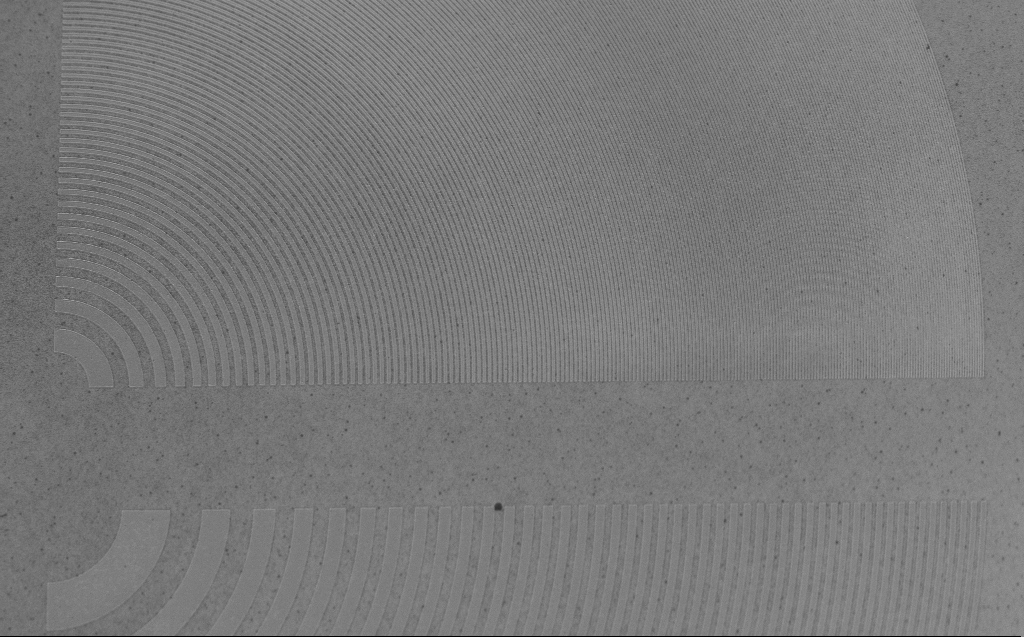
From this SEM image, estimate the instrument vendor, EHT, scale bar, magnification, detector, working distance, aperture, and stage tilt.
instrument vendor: Zeiss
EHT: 5 kV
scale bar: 10000 nm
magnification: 4.62 K X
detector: InLens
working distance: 4 mm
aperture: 30 µm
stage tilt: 30°